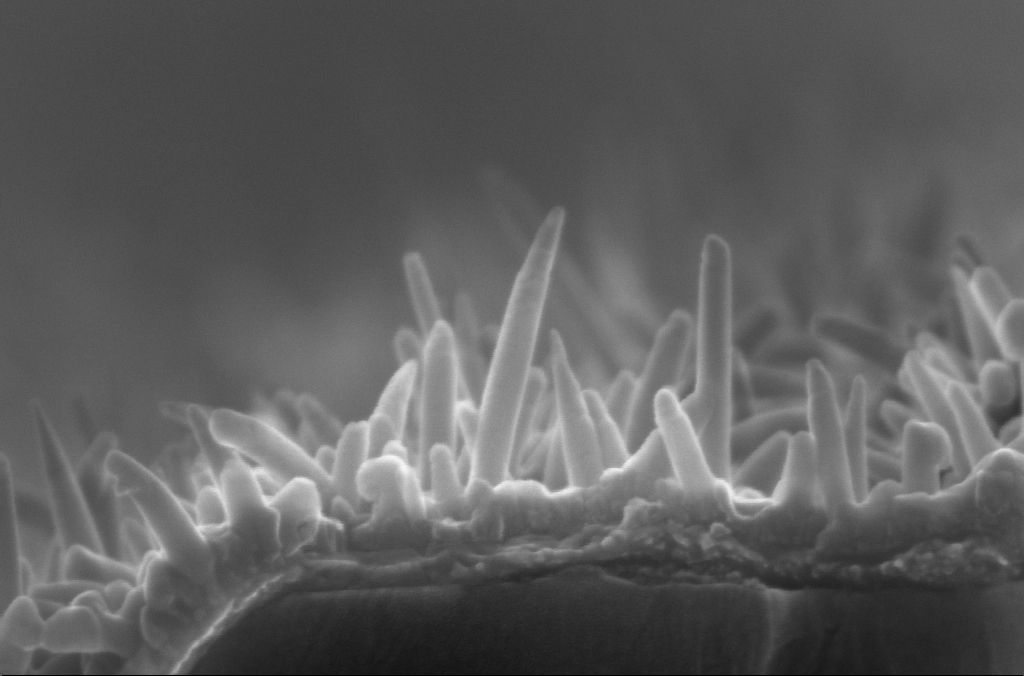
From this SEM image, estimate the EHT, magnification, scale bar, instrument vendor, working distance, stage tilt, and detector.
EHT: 10 kV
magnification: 219.19 K X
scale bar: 100 nm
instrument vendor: Zeiss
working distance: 2.6 mm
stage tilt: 4°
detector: InLens